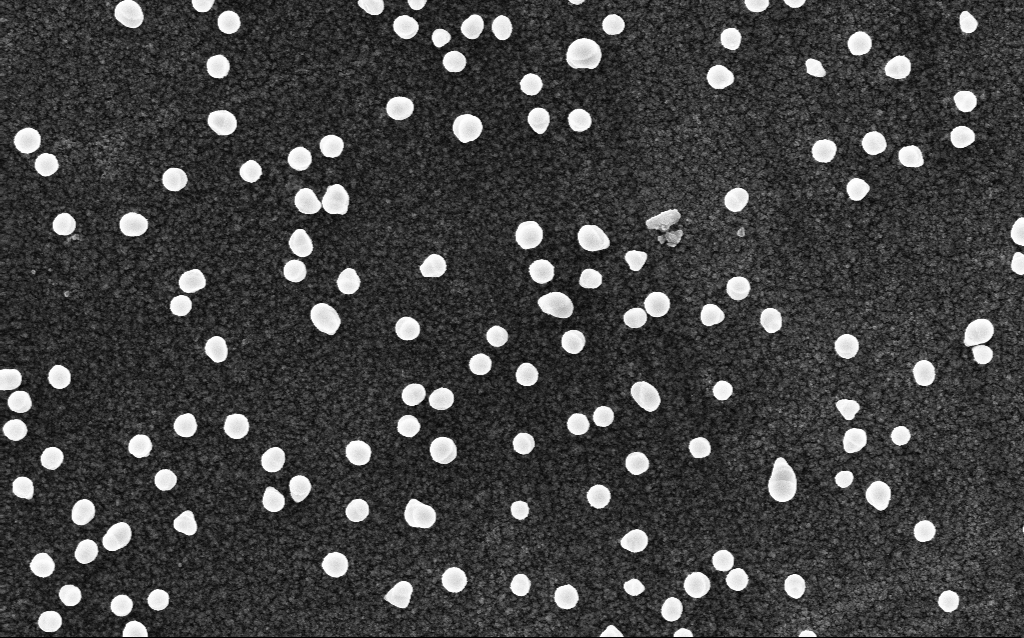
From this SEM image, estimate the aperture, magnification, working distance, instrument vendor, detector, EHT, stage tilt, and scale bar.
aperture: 30 µm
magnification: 50 K X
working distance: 2.8 mm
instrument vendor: Zeiss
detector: InLens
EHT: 5 kV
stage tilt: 0°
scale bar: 1000 nm